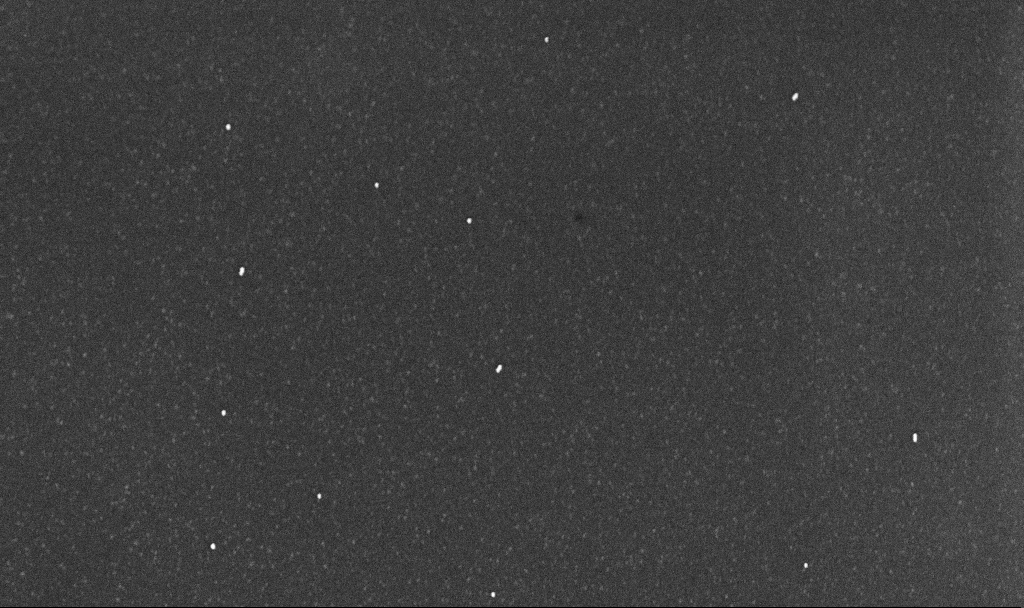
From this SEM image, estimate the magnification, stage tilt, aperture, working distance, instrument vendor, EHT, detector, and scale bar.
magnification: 54.71 K X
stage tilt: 0°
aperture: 30 µm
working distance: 3.1 mm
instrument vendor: Zeiss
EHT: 10 kV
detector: InLens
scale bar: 1000 nm